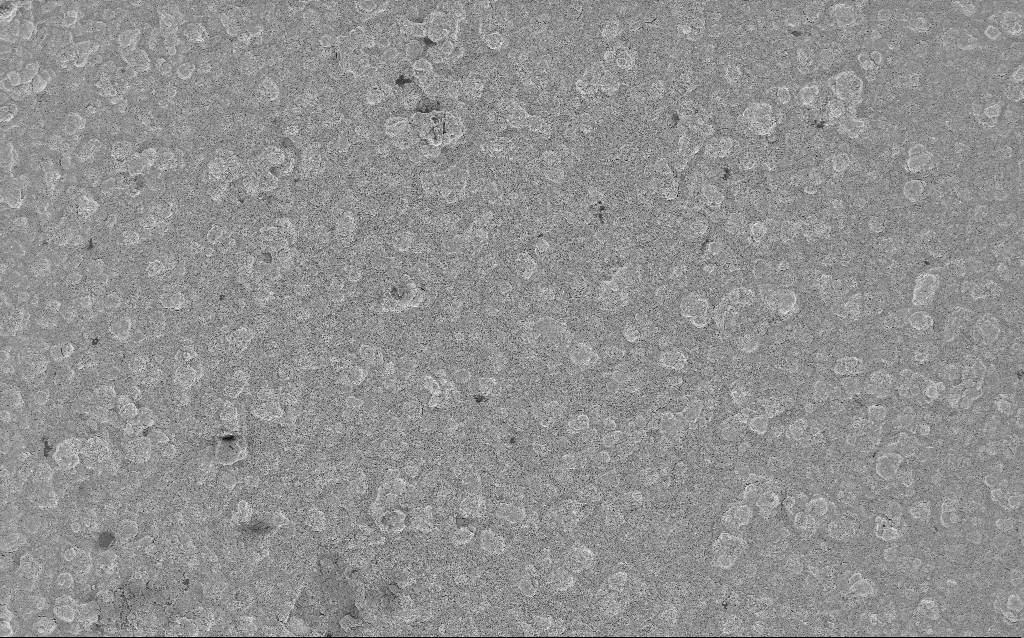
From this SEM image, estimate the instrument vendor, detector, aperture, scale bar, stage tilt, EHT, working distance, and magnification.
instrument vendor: Zeiss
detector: InLens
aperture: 30 µm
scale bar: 20000 nm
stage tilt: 0°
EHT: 3 kV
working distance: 7.6 mm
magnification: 0.77 K X